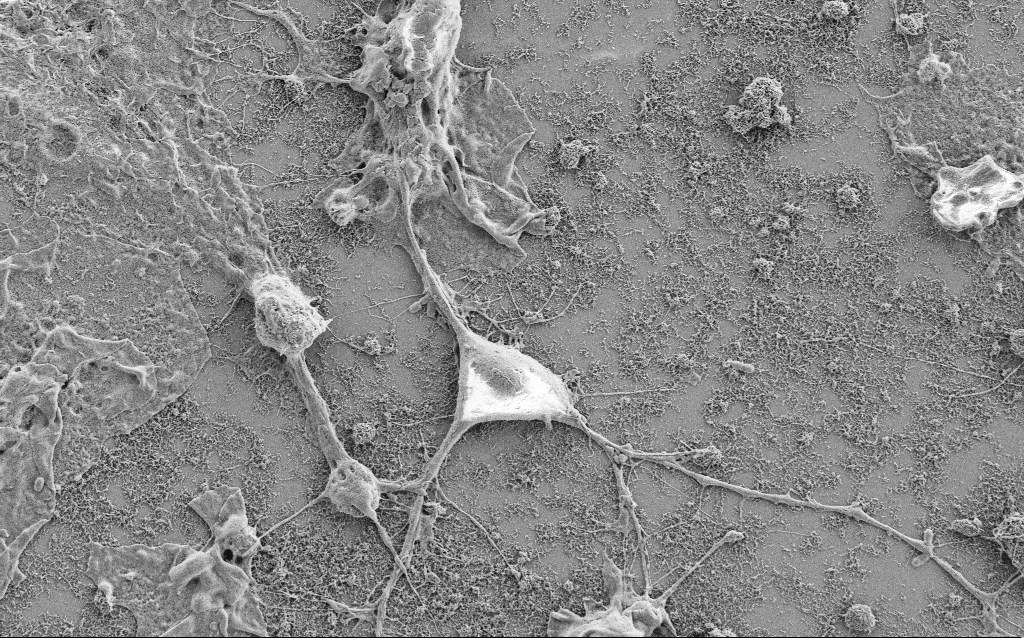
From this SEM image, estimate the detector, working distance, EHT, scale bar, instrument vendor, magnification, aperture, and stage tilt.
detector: SE2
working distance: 6.8 mm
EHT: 2 kV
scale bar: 10000 nm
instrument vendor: Zeiss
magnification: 5 K X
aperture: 30 µm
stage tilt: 0°